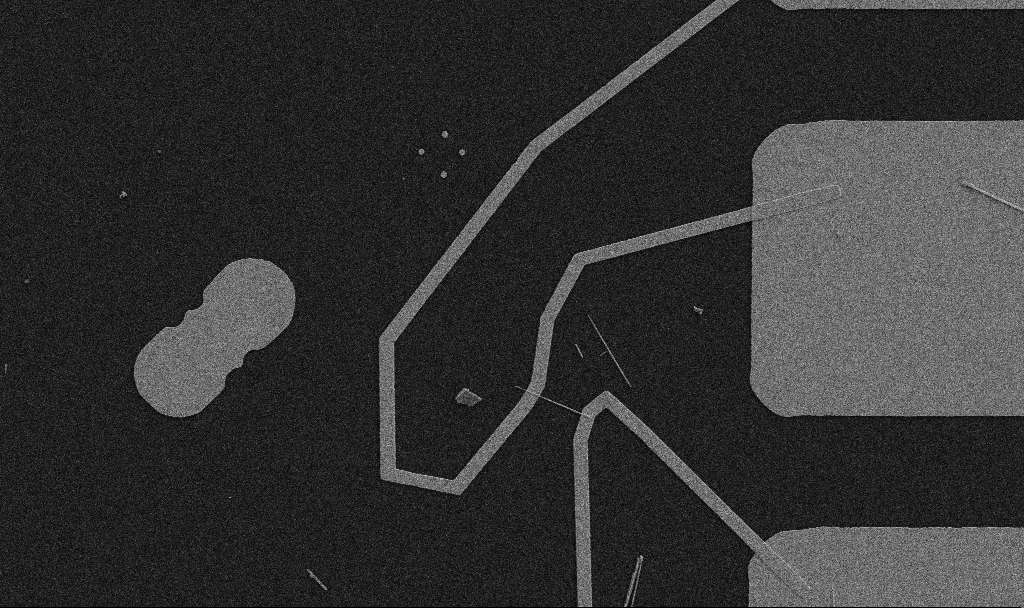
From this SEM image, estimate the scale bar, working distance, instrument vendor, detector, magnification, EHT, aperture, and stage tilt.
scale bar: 10000 nm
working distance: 10.7 mm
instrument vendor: Zeiss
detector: SE2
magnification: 5 K X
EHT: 5 kV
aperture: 30 µm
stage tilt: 0°